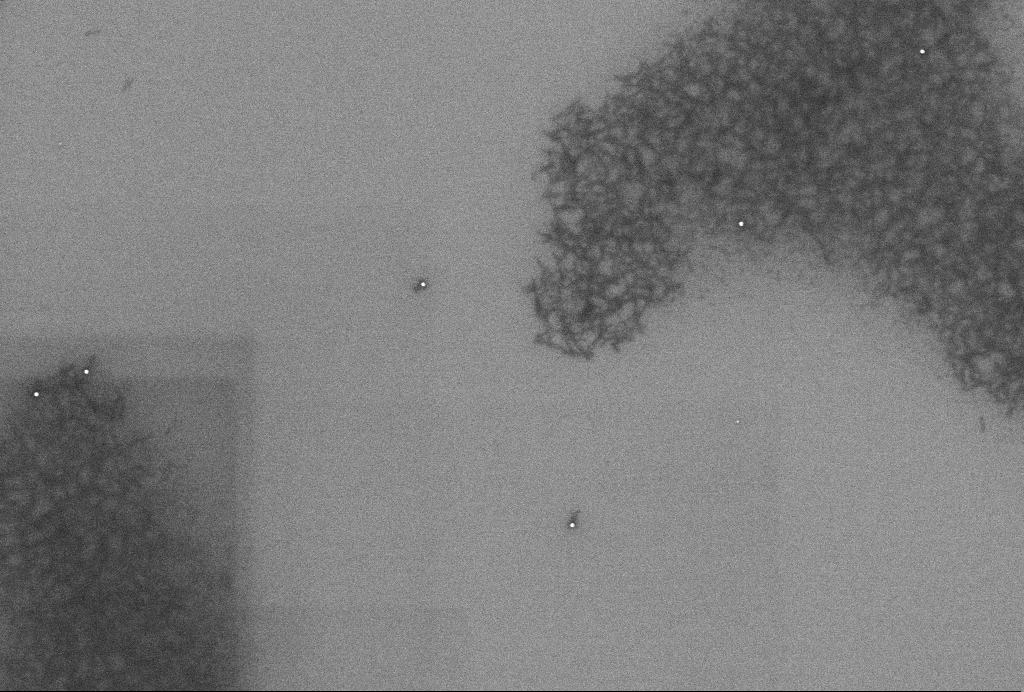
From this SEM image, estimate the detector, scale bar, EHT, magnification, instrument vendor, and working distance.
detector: InLens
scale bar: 1000 nm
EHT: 2 kV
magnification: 55.18 K X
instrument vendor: Zeiss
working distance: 3.3 mm